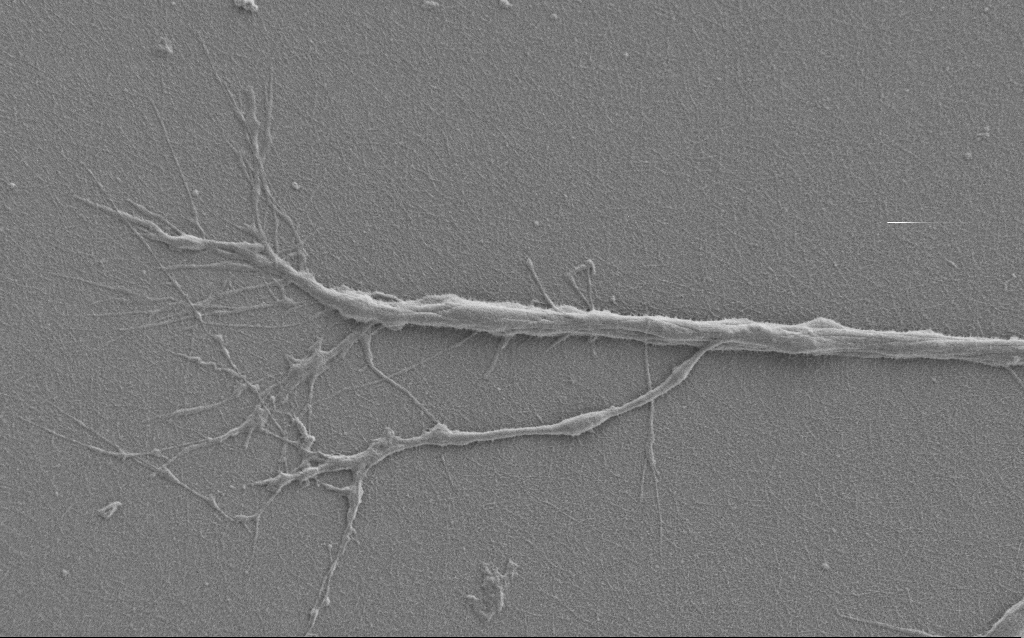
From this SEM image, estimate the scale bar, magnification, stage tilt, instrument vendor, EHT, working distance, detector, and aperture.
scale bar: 10000 nm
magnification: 6 K X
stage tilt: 0°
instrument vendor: Zeiss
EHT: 1 kV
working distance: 6 mm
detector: SE2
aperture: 30 µm